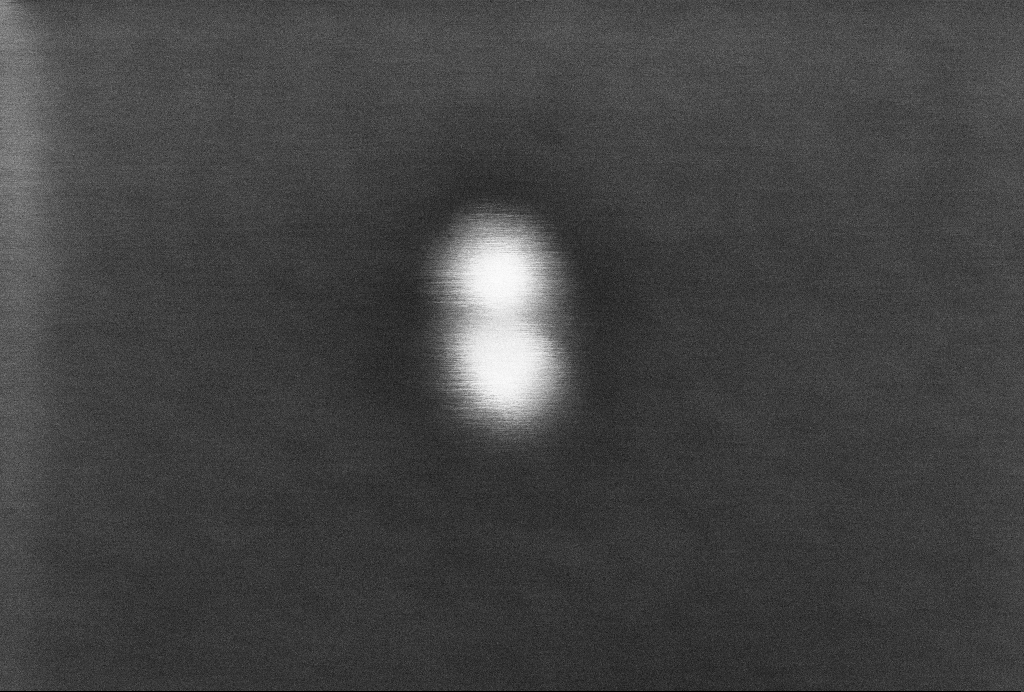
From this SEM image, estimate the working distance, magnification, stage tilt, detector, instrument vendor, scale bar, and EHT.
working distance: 3.3 mm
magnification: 1356.84 K X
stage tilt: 0°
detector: InLens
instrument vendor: Zeiss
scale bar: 20 nm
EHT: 2 kV